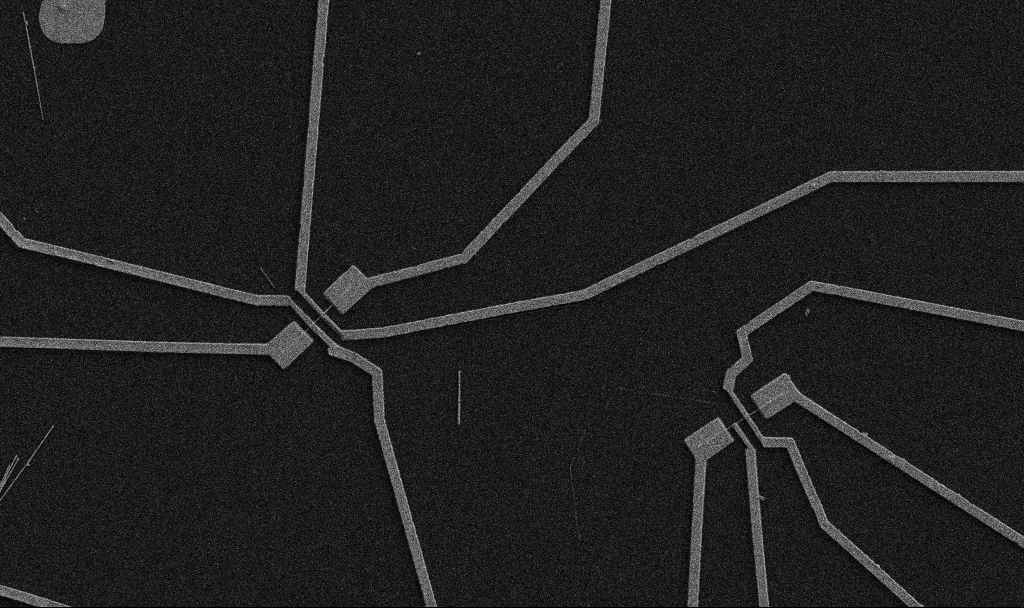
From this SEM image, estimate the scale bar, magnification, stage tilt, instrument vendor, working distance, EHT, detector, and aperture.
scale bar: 10000 nm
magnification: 5 K X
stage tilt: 0°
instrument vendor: Zeiss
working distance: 10.7 mm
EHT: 5 kV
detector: SE2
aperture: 30 µm